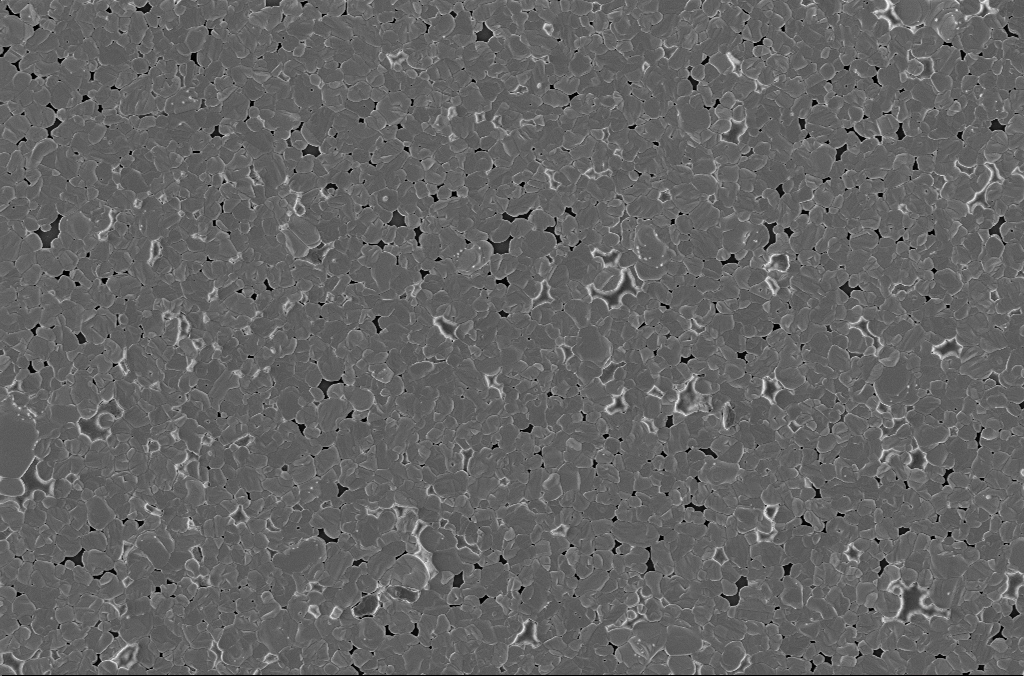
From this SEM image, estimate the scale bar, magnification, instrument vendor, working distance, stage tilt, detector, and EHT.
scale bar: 2000 nm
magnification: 10 K X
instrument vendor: Zeiss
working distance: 3 mm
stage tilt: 0°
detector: InLens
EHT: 2 kV